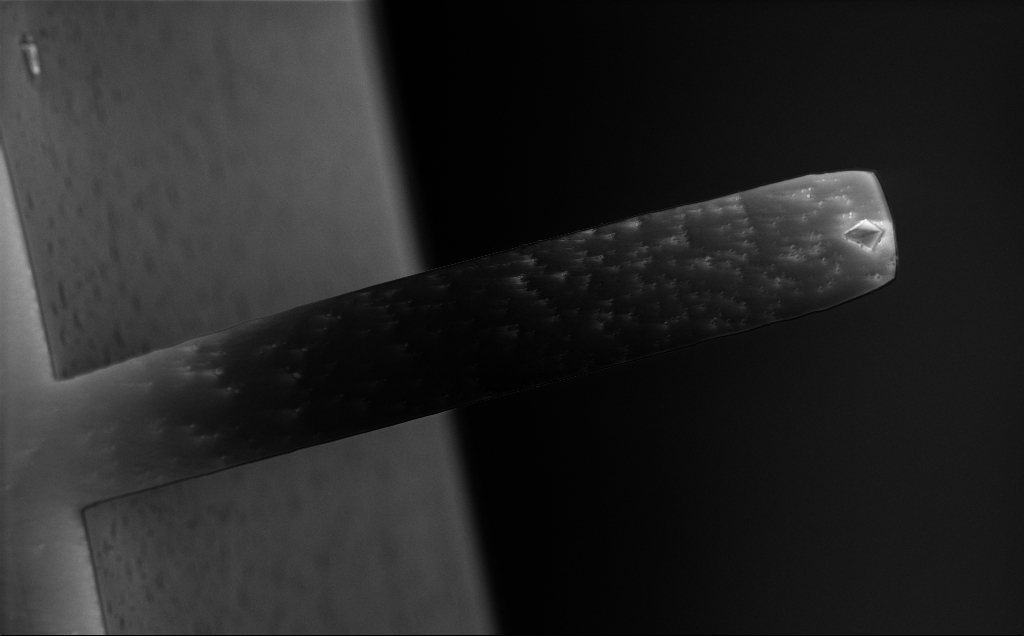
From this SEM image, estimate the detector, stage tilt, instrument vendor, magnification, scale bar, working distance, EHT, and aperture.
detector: InLens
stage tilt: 0°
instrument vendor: Zeiss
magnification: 1.42 K X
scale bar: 20000 nm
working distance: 8 mm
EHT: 5 kV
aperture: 30 µm